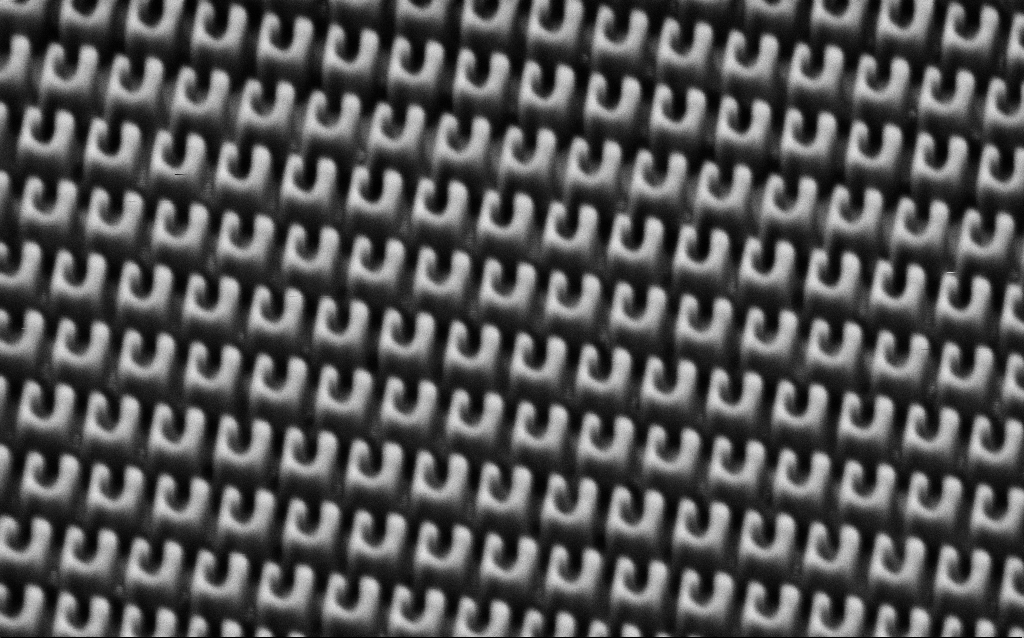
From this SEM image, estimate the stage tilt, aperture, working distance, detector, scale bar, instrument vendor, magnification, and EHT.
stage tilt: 30°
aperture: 30 µm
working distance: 6.7 mm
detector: SE2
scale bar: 1000 nm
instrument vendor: Zeiss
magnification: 52.85 K X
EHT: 1.5 kV